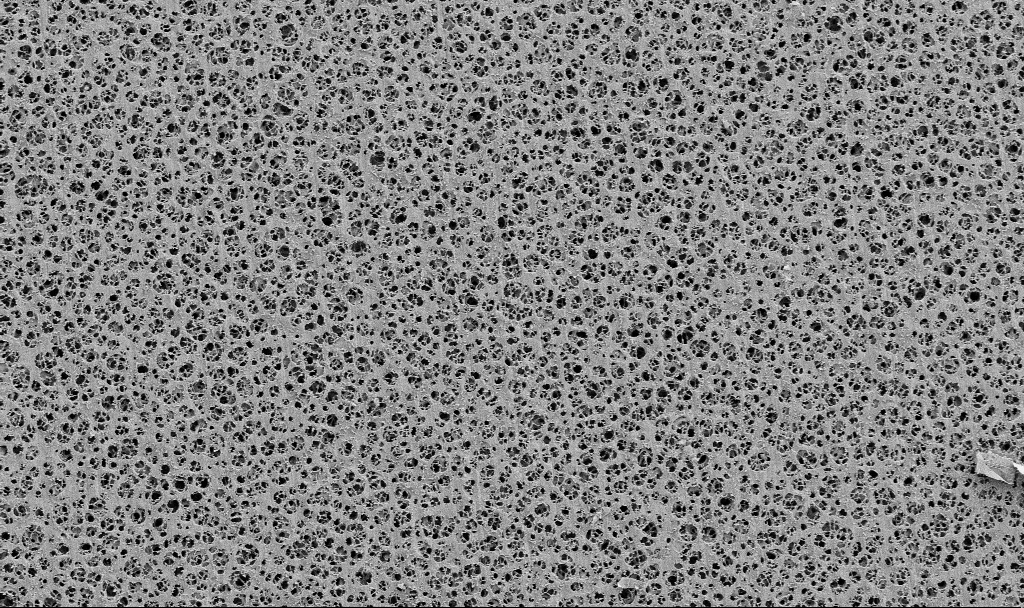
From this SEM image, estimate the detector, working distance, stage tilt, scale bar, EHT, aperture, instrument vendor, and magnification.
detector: SE2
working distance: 3.7 mm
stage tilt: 0°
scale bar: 10000 nm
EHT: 2 kV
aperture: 30 µm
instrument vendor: Zeiss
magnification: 2 K X